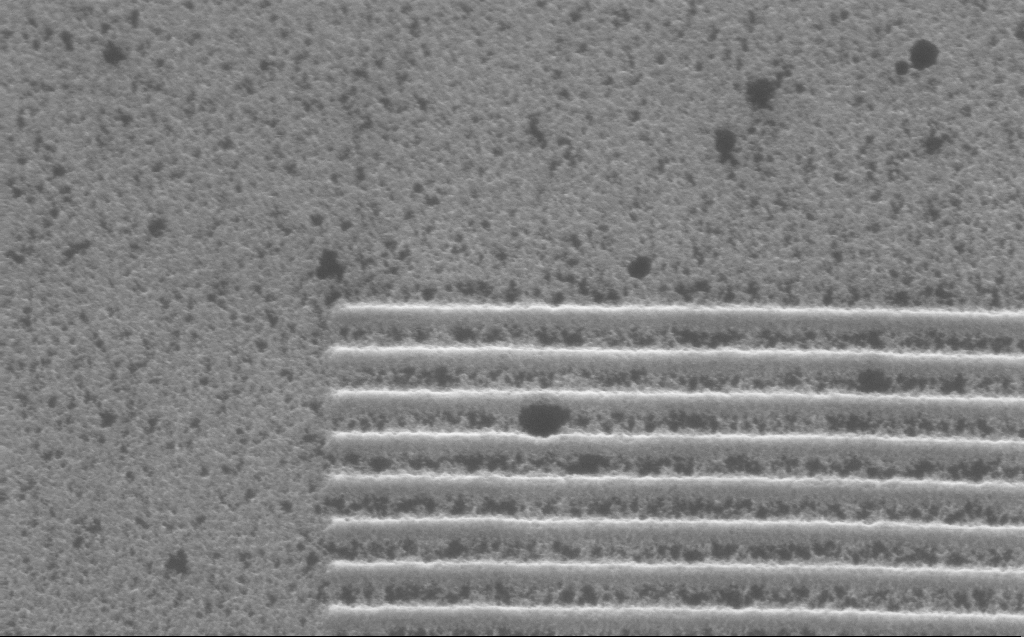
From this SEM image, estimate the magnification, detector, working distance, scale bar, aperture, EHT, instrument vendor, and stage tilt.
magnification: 76.75 K X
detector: InLens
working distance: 4 mm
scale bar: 200 nm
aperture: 30 µm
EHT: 5 kV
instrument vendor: Zeiss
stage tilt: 30°